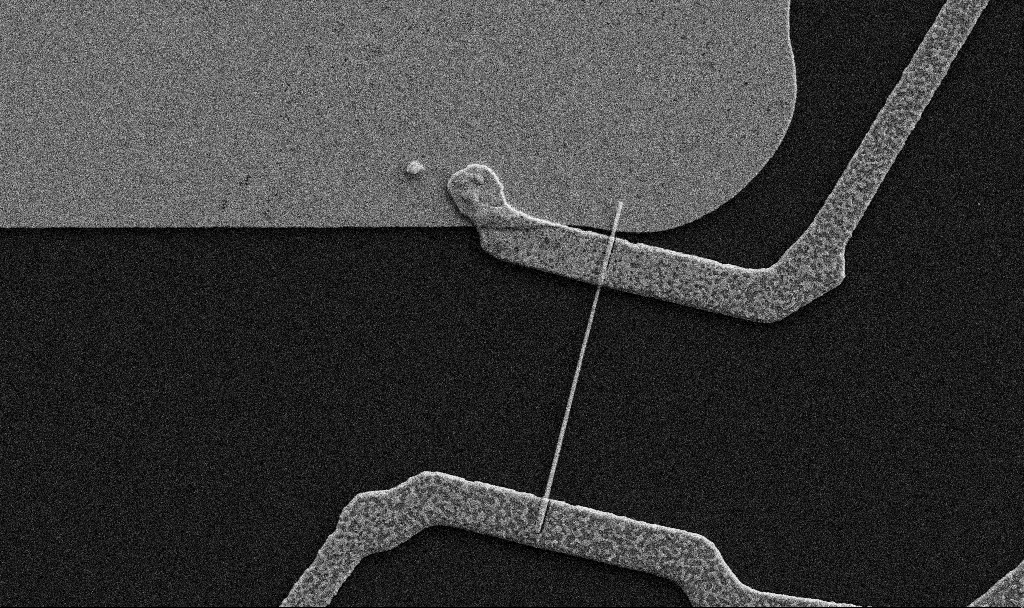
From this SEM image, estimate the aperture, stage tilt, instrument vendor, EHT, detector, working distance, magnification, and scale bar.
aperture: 30 µm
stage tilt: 0°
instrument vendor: Zeiss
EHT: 5 kV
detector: SE2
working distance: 6.7 mm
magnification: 20 K X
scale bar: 1000 nm